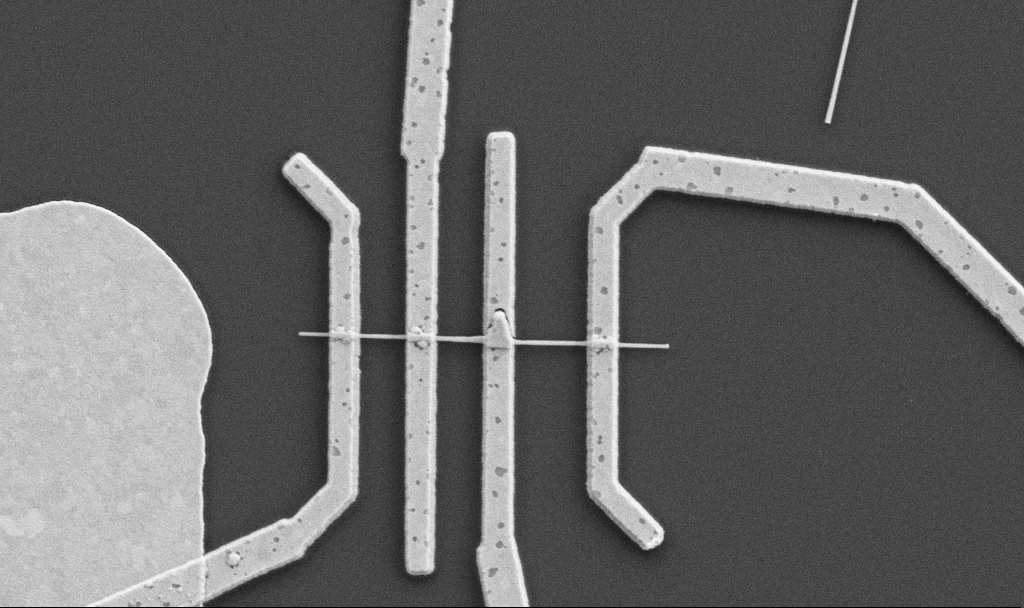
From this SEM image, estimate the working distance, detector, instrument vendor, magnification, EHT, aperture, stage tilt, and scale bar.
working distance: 10.6 mm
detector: SE2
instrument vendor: Zeiss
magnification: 20 K X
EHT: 5 kV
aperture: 30 µm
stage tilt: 0°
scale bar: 2000 nm